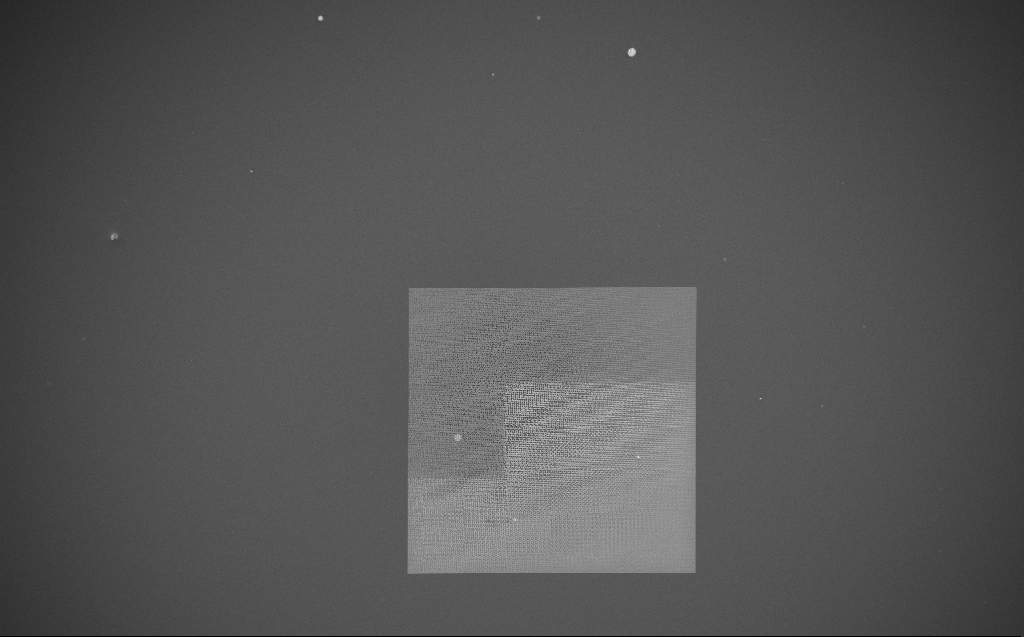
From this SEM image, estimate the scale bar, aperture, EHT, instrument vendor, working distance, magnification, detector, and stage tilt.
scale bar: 100000 nm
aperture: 30 µm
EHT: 5 kV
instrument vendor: Zeiss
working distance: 7 mm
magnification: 0.143 K X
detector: InLens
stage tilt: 0°